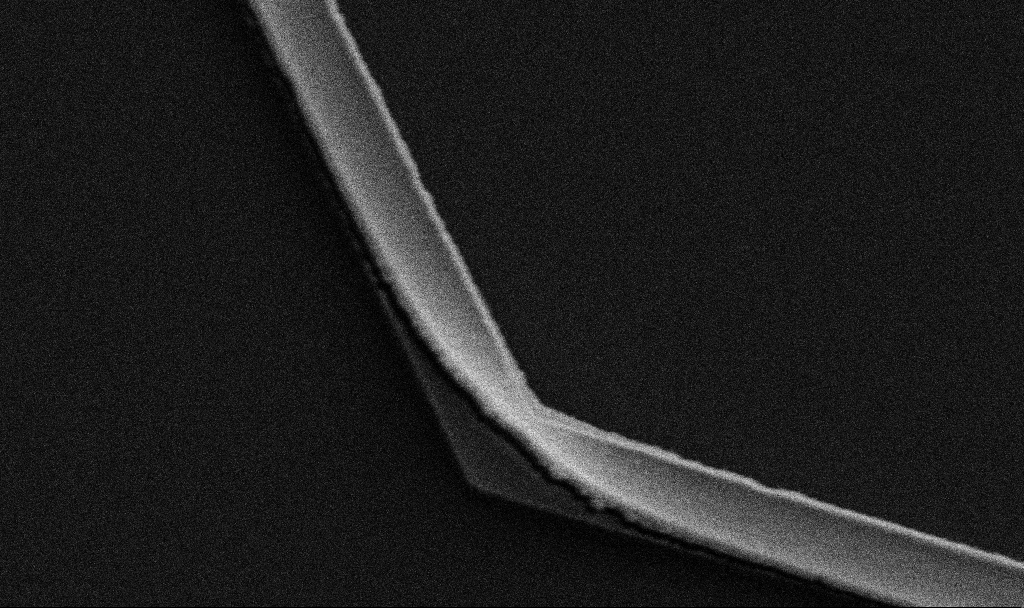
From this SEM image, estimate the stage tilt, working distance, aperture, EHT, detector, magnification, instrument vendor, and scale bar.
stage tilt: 0°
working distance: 10.7 mm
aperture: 30 µm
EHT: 5 kV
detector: SE2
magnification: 32.72 K X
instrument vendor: Zeiss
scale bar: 2000 nm